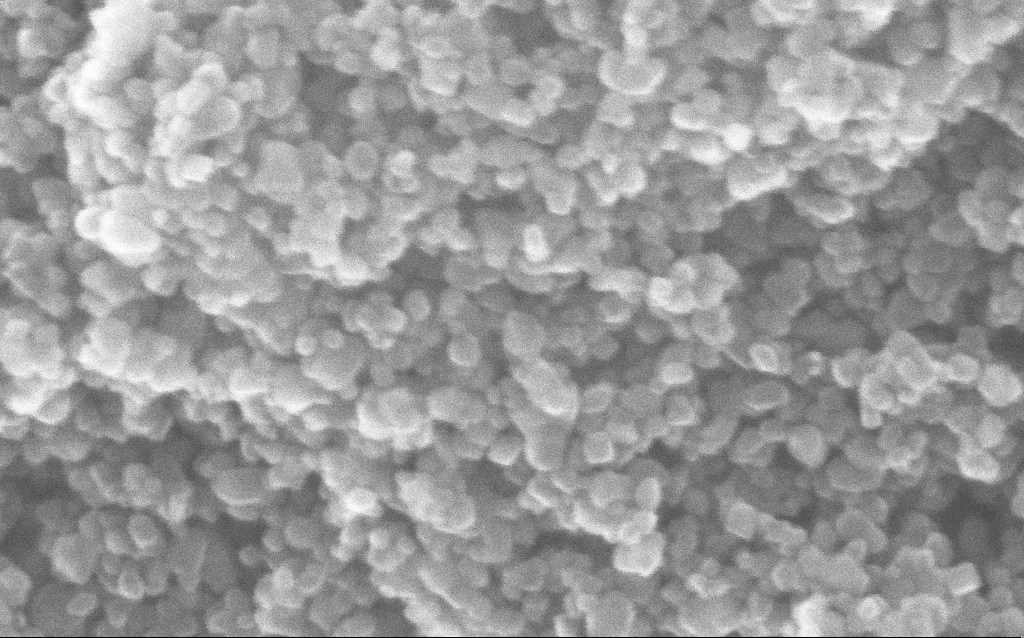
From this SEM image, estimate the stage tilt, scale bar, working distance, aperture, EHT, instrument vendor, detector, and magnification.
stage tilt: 0°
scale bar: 100 nm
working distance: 3.6 mm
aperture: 30 µm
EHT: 10 kV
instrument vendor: Zeiss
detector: InLens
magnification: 440.06 K X